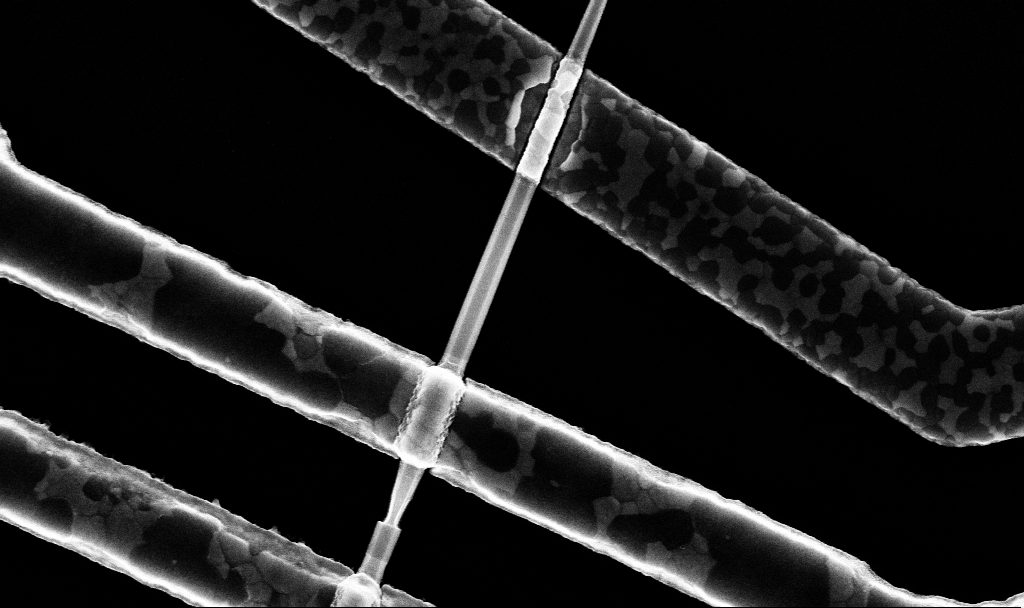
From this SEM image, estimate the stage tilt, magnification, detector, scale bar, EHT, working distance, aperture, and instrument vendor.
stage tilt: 0°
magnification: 64 K X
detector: InLens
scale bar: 1000 nm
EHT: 10 kV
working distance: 7.7 mm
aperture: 30 µm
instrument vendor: Zeiss